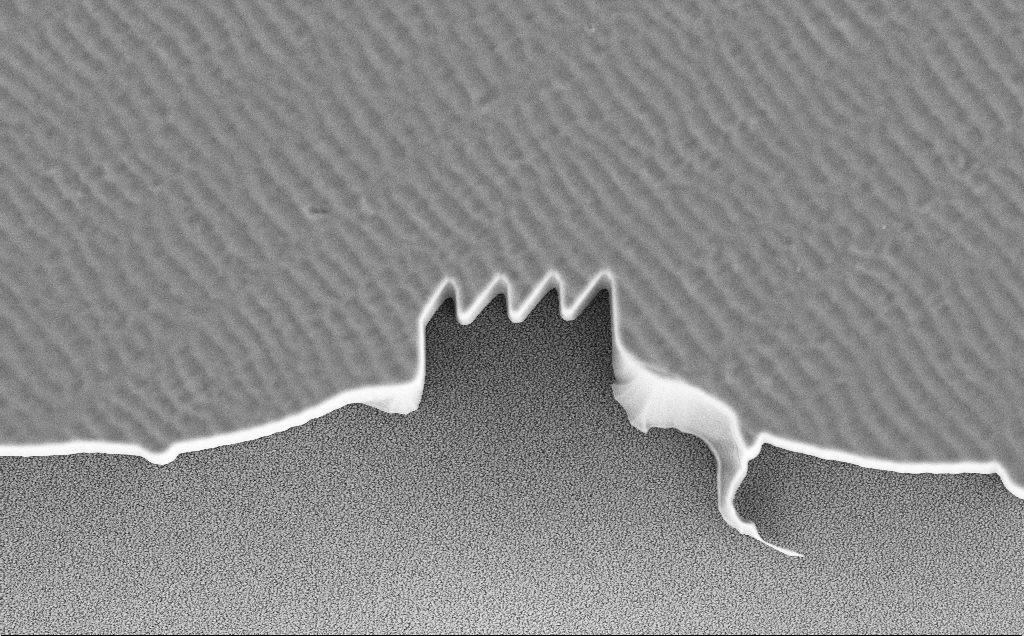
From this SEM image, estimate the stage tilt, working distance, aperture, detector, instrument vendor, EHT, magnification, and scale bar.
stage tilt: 0°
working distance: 13 mm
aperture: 30 µm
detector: SE2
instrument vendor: Zeiss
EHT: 10 kV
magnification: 1.65 K X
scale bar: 20000 nm